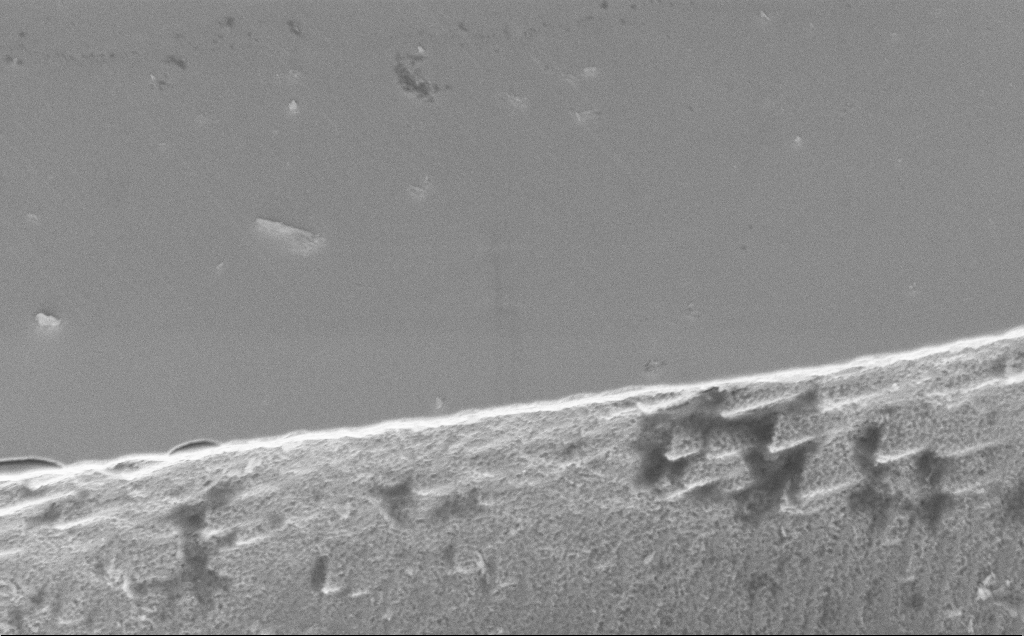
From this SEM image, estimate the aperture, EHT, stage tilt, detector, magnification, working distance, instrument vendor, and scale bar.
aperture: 30 µm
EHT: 5 kV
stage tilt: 45°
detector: InLens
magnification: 12.12 K X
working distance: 10 mm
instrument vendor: Zeiss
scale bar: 2000 nm